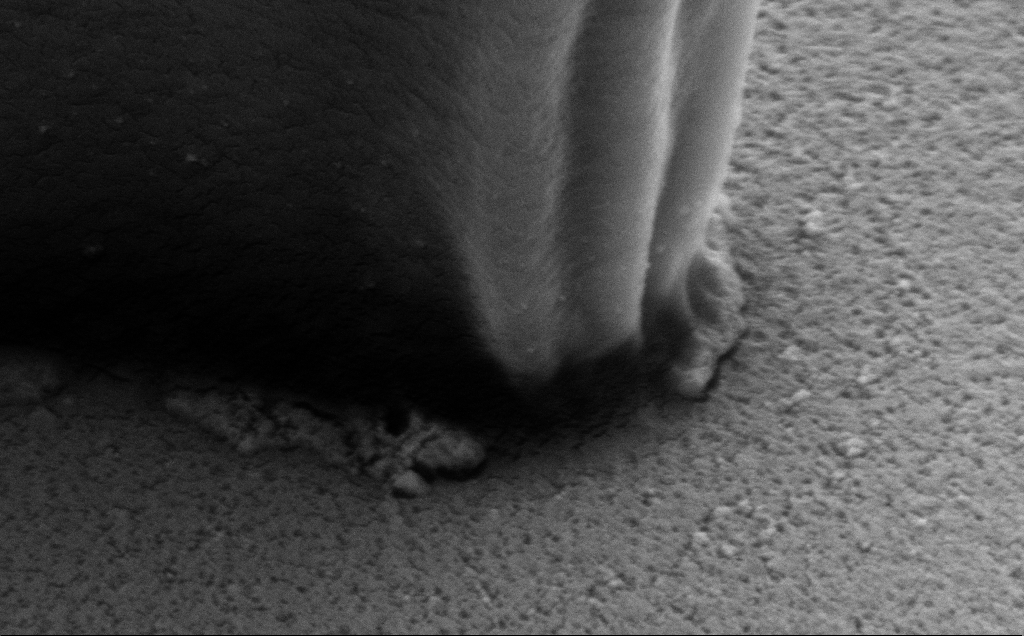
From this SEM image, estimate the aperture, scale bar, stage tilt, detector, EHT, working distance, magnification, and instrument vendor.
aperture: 30 µm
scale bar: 200 nm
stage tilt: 40°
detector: SE2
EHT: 5 kV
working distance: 8 mm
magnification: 105.81 K X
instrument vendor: Zeiss